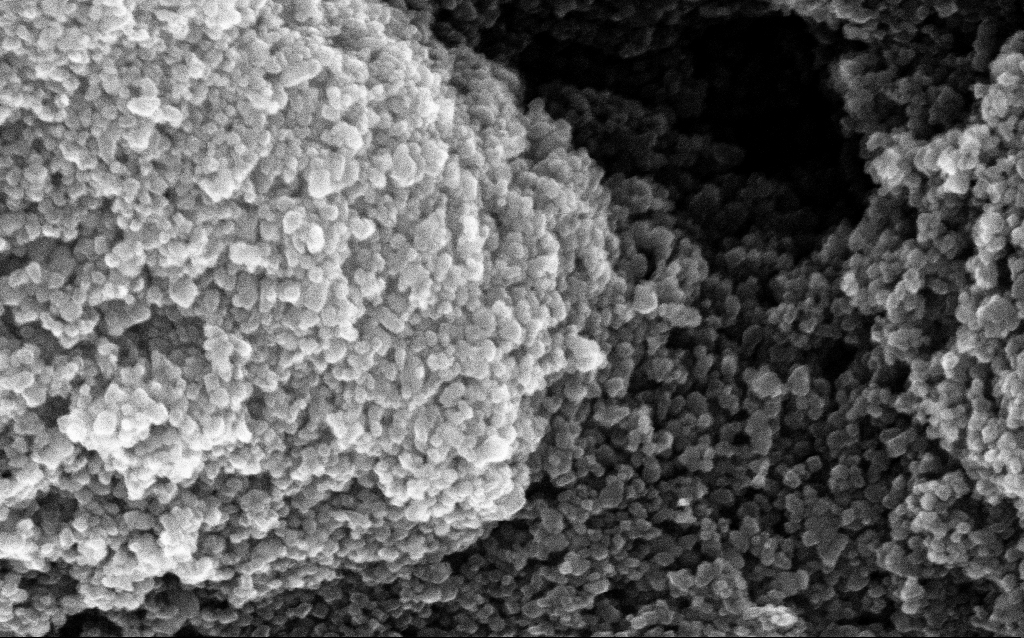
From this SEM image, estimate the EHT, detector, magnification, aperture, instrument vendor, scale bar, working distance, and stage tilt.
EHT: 10 kV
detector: InLens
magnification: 220.1 K X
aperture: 30 µm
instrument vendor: Zeiss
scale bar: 200 nm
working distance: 1.8 mm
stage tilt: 0°